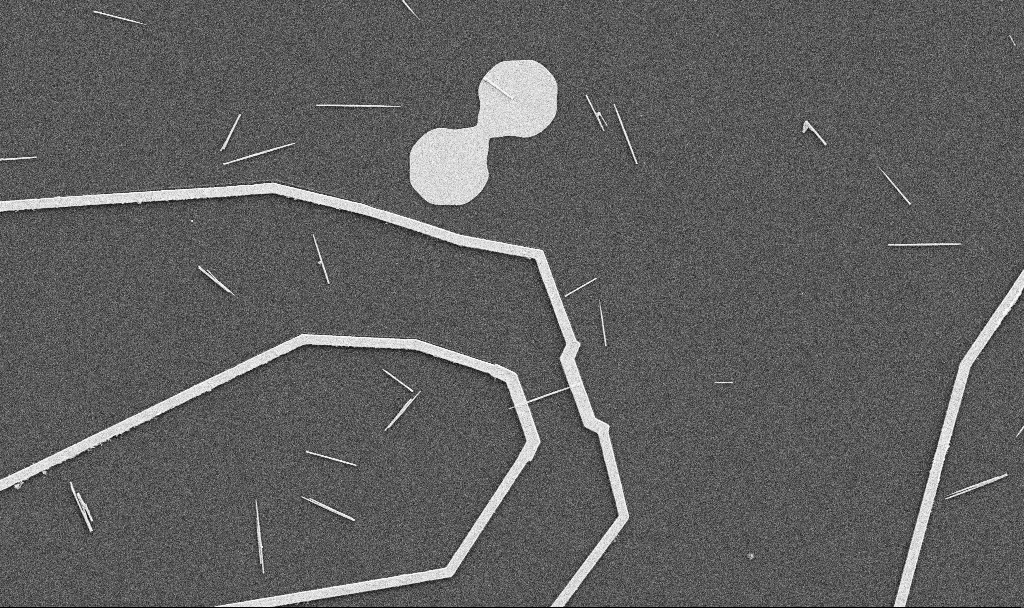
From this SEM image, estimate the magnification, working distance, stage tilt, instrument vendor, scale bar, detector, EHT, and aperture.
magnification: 5 K X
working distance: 10.7 mm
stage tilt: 0°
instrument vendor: Zeiss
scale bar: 10000 nm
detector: SE2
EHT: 5 kV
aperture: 30 µm